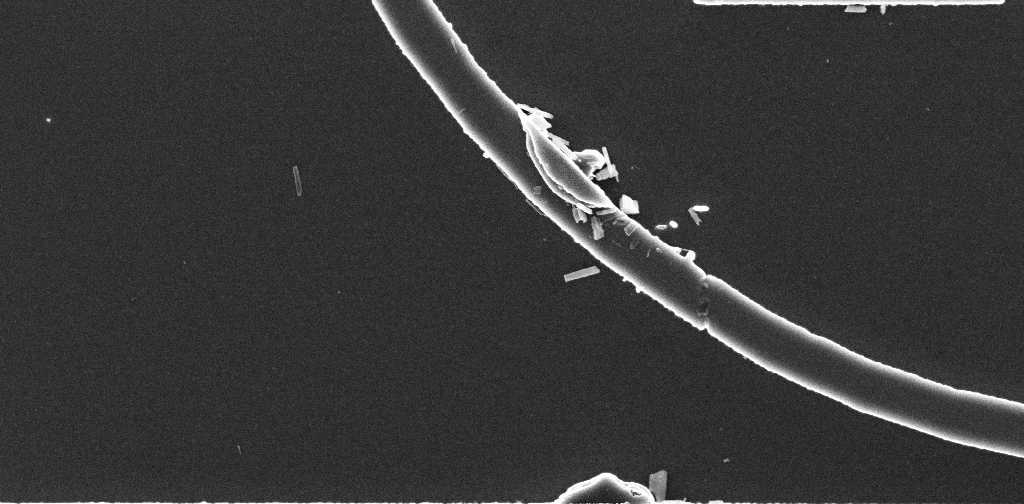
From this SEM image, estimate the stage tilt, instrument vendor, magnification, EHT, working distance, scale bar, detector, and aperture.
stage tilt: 0°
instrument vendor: Zeiss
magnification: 38.41 K X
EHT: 3 kV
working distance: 2.9 mm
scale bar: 1000 nm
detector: InLens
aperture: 30 µm